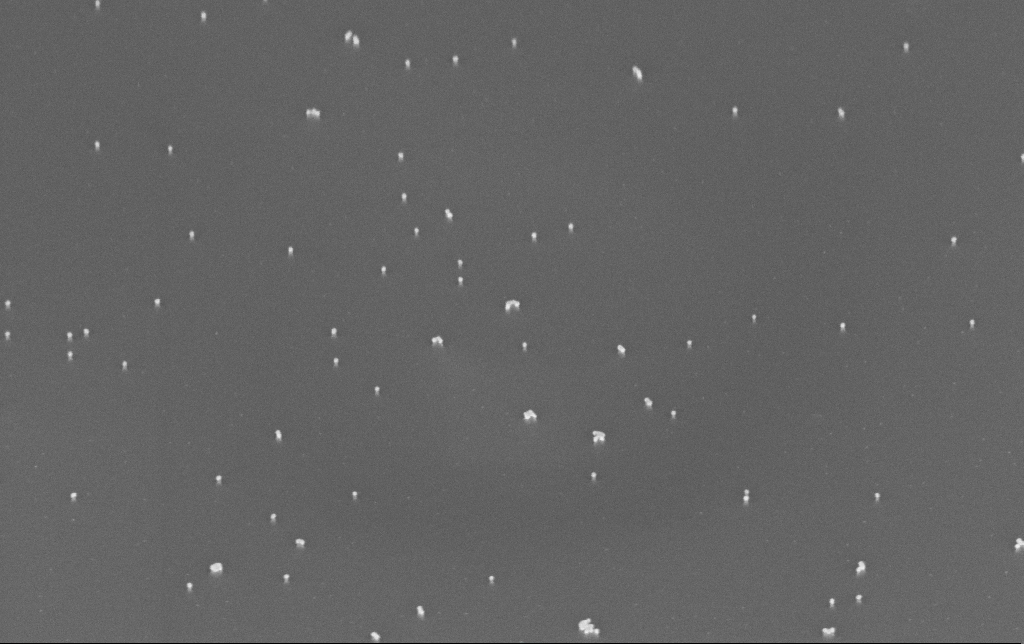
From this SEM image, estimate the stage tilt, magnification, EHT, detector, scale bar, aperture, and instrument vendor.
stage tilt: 45°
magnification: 100 K X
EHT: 10 kV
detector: InLens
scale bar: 200 nm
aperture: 30 µm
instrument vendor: Zeiss